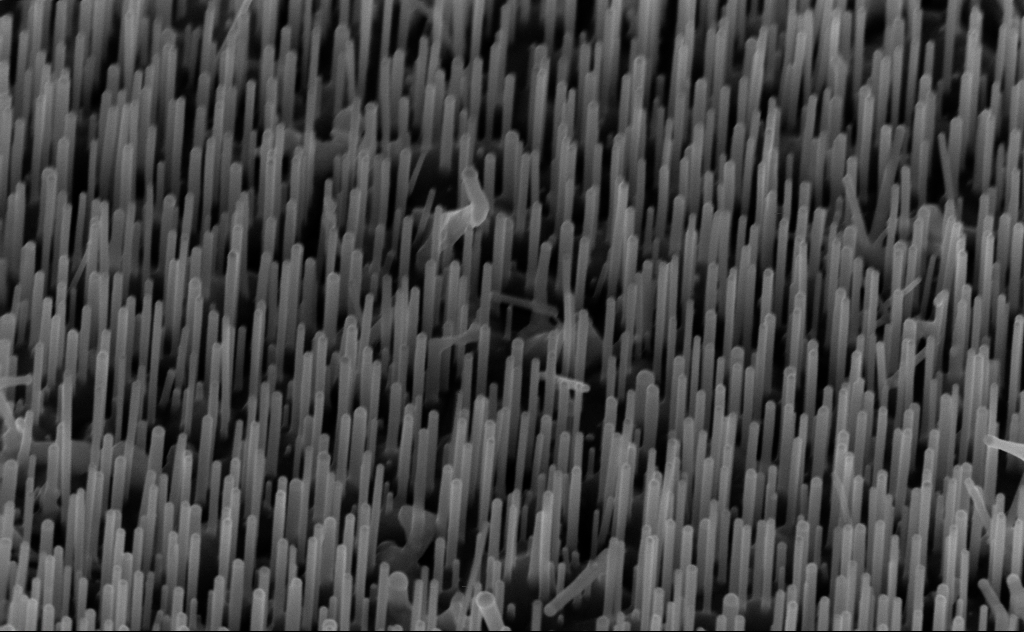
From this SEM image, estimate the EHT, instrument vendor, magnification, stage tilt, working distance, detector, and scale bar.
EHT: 10 kV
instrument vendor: Zeiss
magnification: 80 K X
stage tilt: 45°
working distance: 6 mm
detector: InLens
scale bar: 200 nm